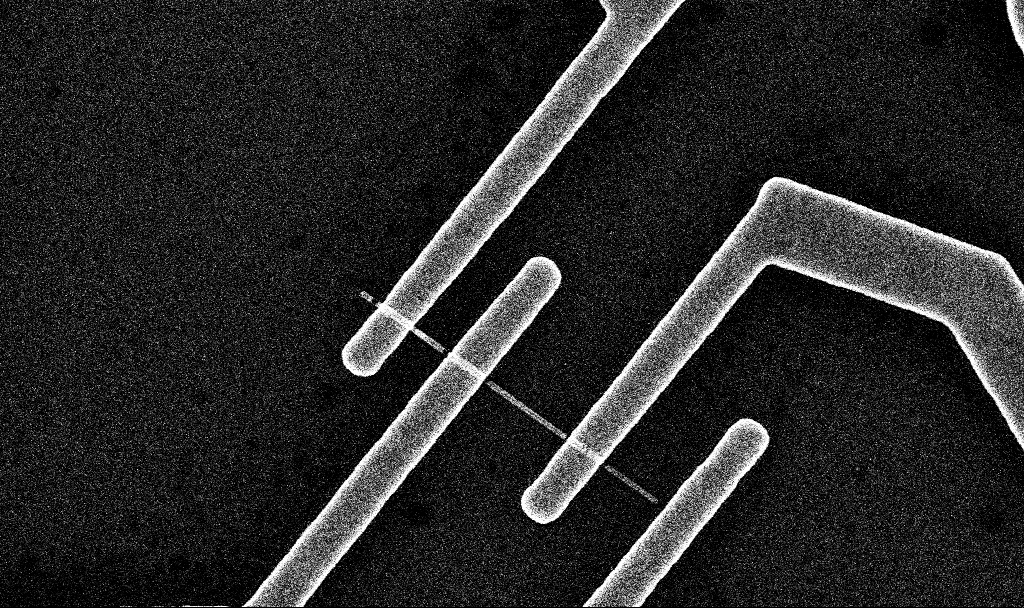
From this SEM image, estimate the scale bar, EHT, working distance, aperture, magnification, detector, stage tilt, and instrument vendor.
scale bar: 1000 nm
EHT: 10 kV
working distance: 7 mm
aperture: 30 µm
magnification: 36.58 K X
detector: InLens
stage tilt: -0°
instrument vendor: Zeiss